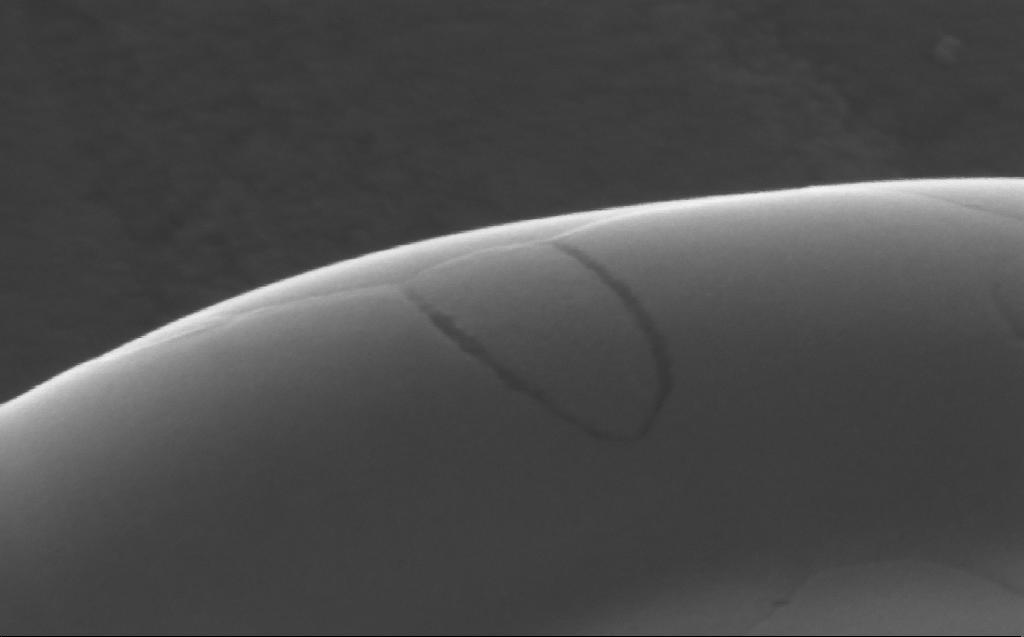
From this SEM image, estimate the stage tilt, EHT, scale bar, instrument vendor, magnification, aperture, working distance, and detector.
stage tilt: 24.9°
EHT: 5 kV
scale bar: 200 nm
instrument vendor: Zeiss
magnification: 286.3 K X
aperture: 30 µm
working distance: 4 mm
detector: InLens